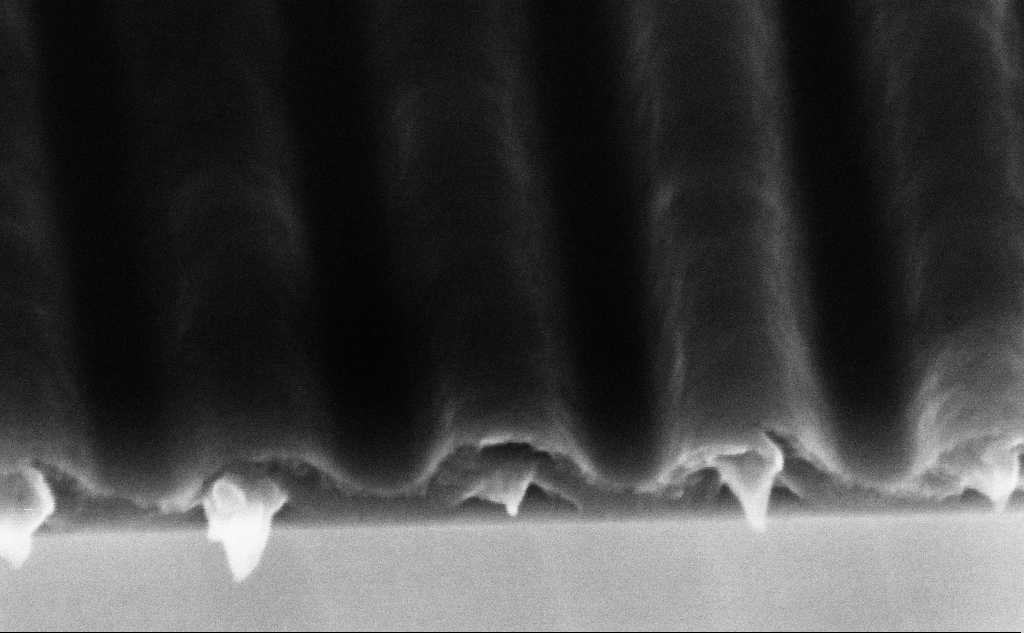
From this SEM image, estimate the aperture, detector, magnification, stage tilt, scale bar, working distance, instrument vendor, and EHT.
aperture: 30 µm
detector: InLens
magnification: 302.05 K X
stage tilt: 45°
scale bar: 100 nm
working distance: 7.4 mm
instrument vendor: Zeiss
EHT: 5 kV